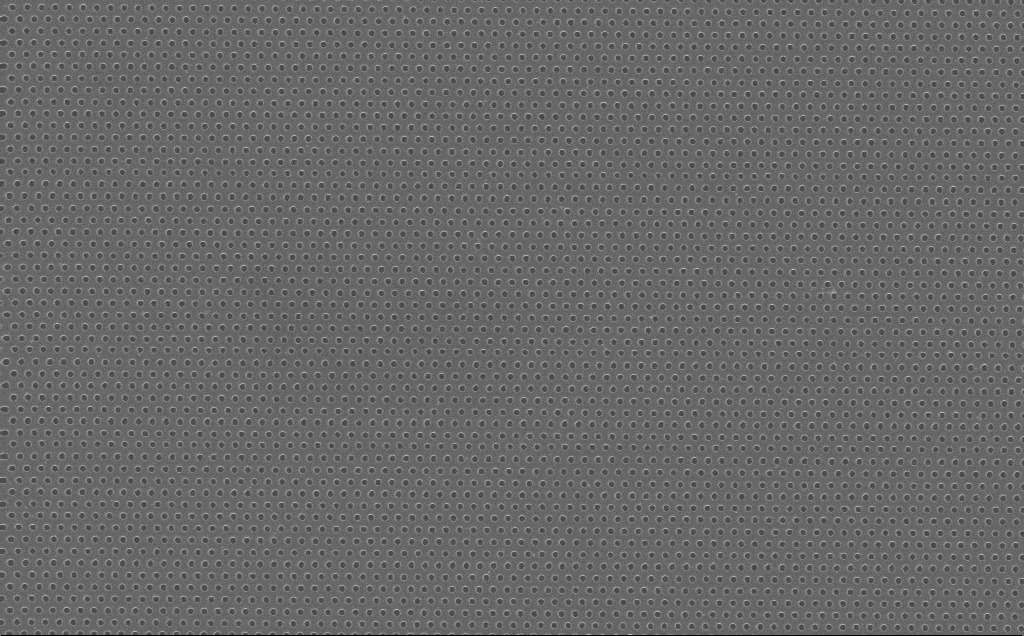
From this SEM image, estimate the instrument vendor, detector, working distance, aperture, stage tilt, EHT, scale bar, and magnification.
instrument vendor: Zeiss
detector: InLens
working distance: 7 mm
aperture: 30 µm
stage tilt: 0°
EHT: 10 kV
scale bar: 2000 nm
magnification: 20.27 K X